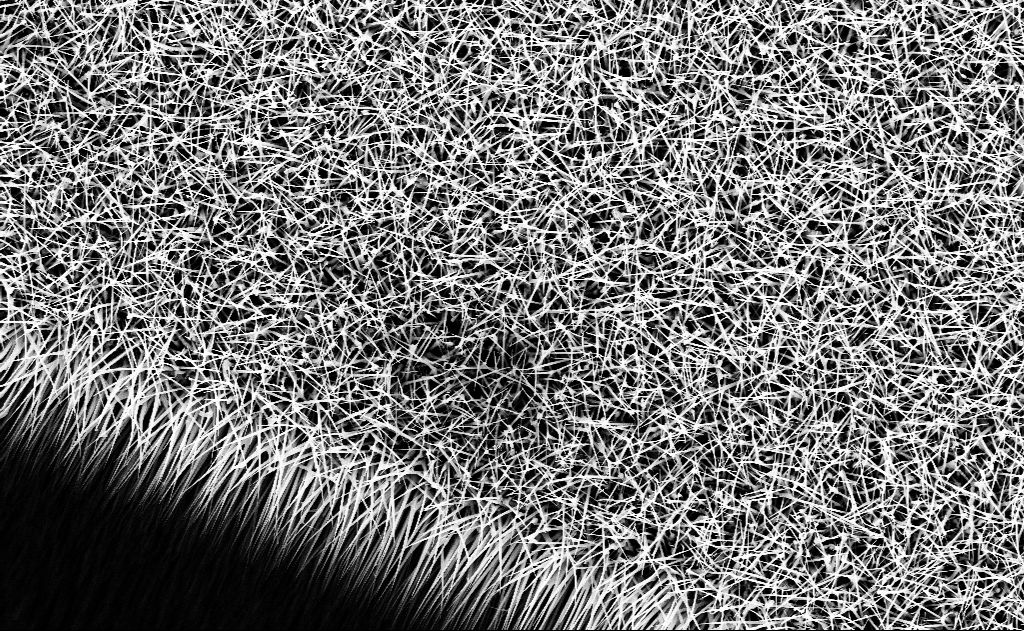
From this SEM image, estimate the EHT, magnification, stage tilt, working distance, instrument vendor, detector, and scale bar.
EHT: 10 kV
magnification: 10 K X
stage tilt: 0°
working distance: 13 mm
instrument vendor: Zeiss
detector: InLens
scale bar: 2000 nm